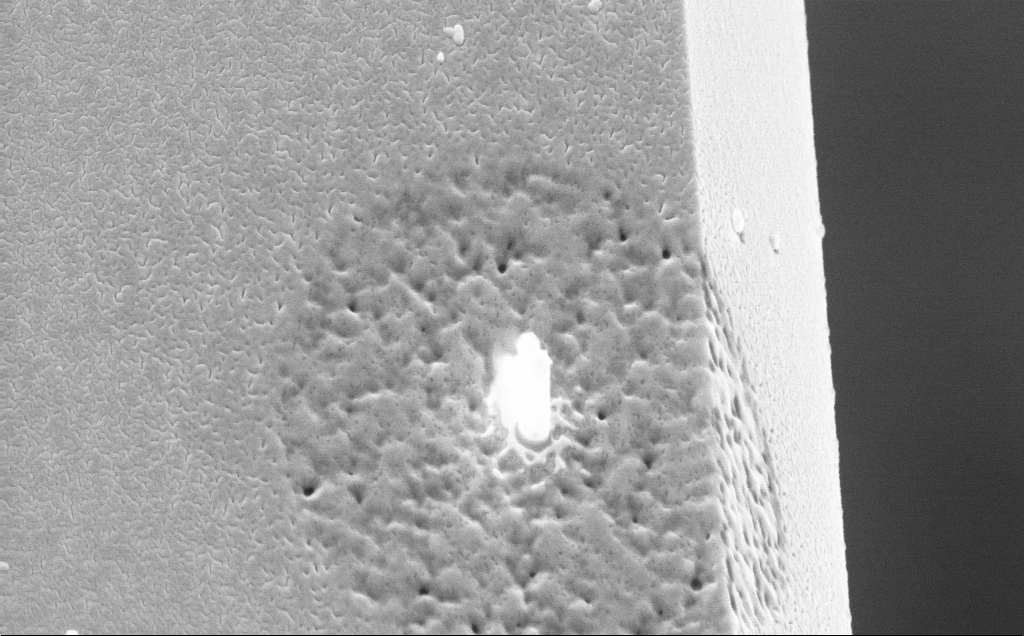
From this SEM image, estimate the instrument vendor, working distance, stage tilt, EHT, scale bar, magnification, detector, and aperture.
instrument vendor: Zeiss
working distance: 3 mm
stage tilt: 44.2°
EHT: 10 kV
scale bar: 1000 nm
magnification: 38.19 K X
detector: InLens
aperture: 30 µm